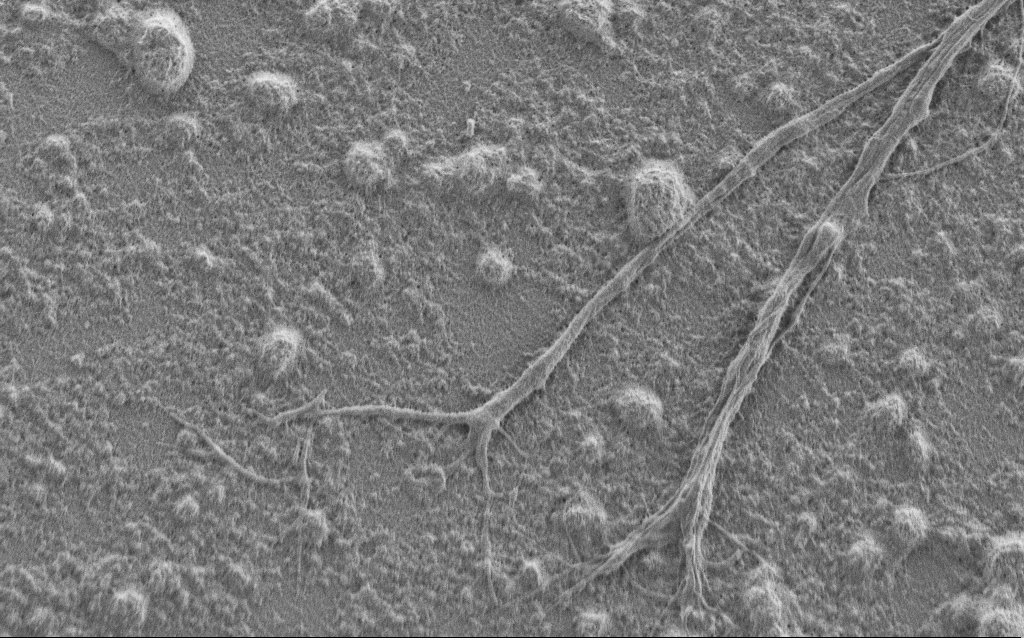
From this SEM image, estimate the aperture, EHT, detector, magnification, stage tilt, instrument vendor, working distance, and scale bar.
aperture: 30 µm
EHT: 1 kV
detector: SE2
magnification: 7.5 K X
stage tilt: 0°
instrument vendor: Zeiss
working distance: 6 mm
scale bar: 2000 nm